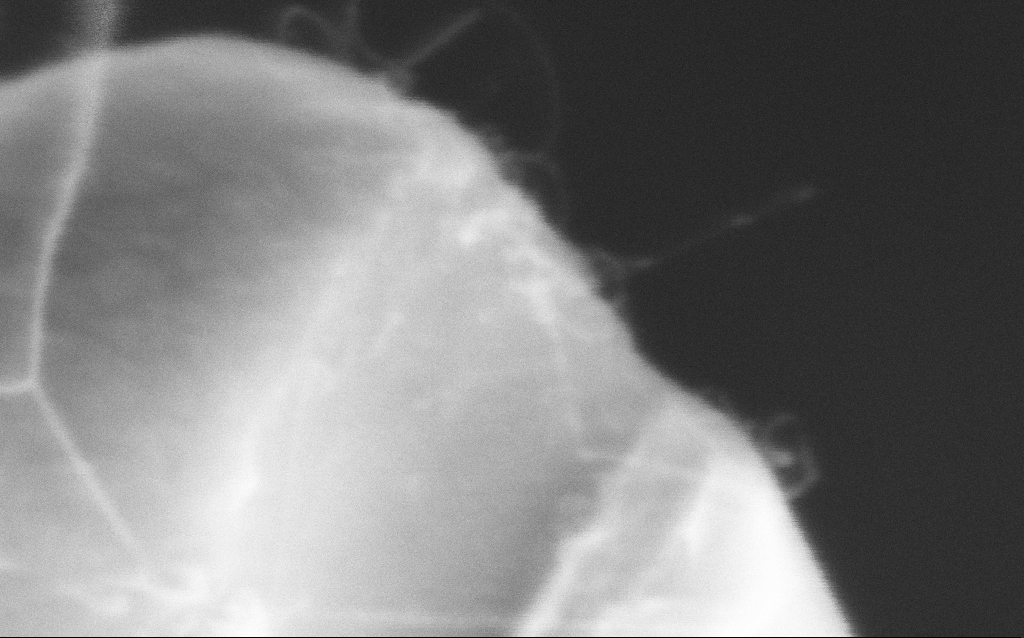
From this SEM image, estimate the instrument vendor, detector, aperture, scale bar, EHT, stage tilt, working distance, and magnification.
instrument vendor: Zeiss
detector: InLens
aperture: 30 µm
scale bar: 100 nm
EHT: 5 kV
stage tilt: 42.7°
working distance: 9 mm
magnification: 553 K X